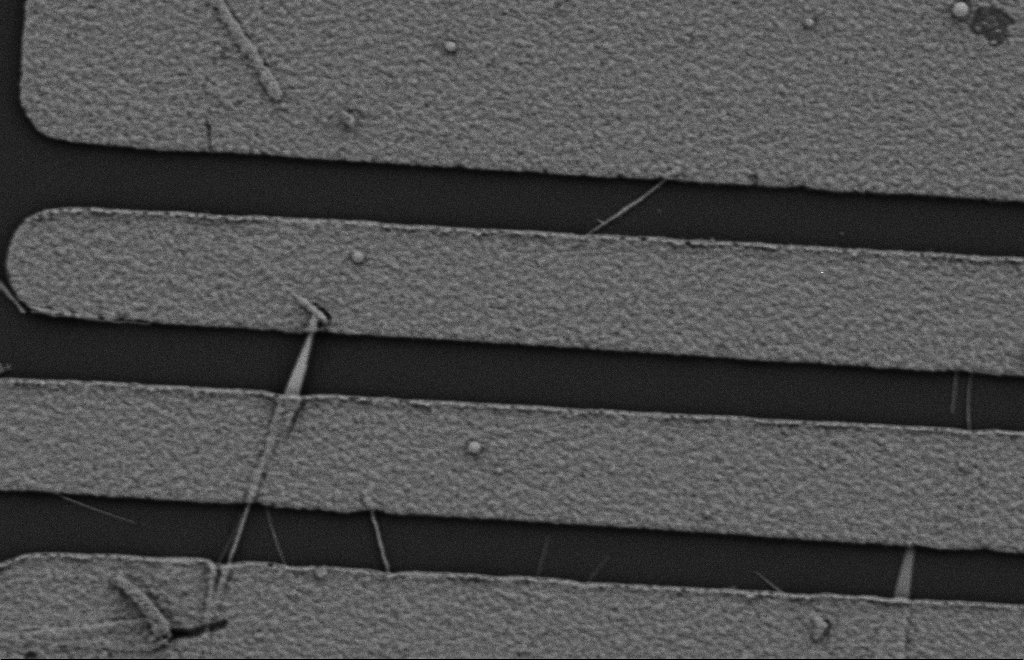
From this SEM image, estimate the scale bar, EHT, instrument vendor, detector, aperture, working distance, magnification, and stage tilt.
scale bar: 2000 nm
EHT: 2 kV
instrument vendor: Zeiss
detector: SE2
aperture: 20 µm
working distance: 10 mm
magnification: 15.8 K X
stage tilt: -0.3°